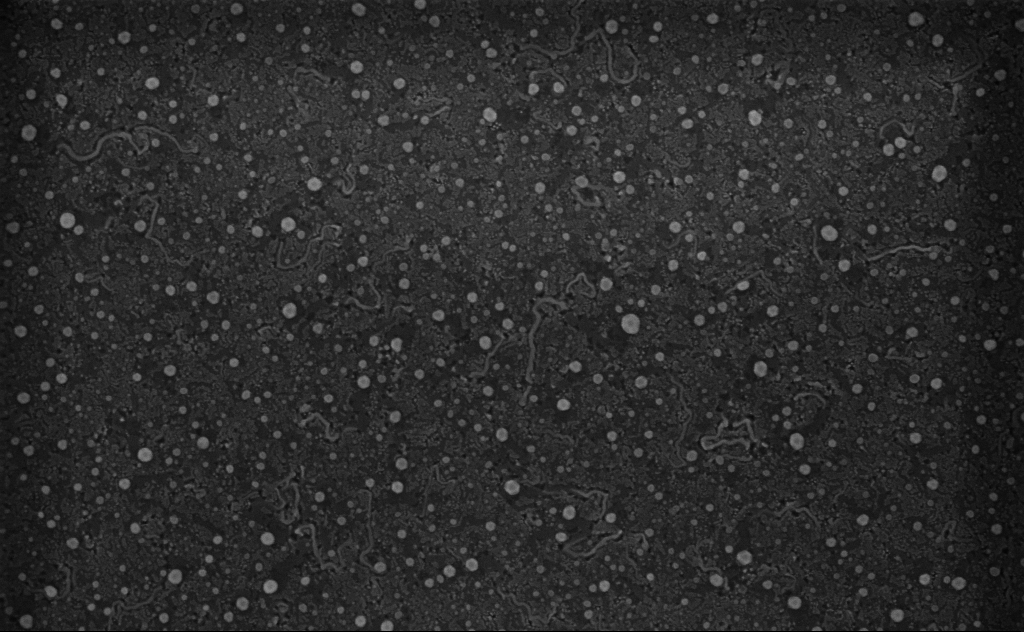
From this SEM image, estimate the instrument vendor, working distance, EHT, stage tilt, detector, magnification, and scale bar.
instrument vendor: Zeiss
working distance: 4 mm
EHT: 3 kV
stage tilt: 0°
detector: InLens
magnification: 80 K X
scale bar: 200 nm